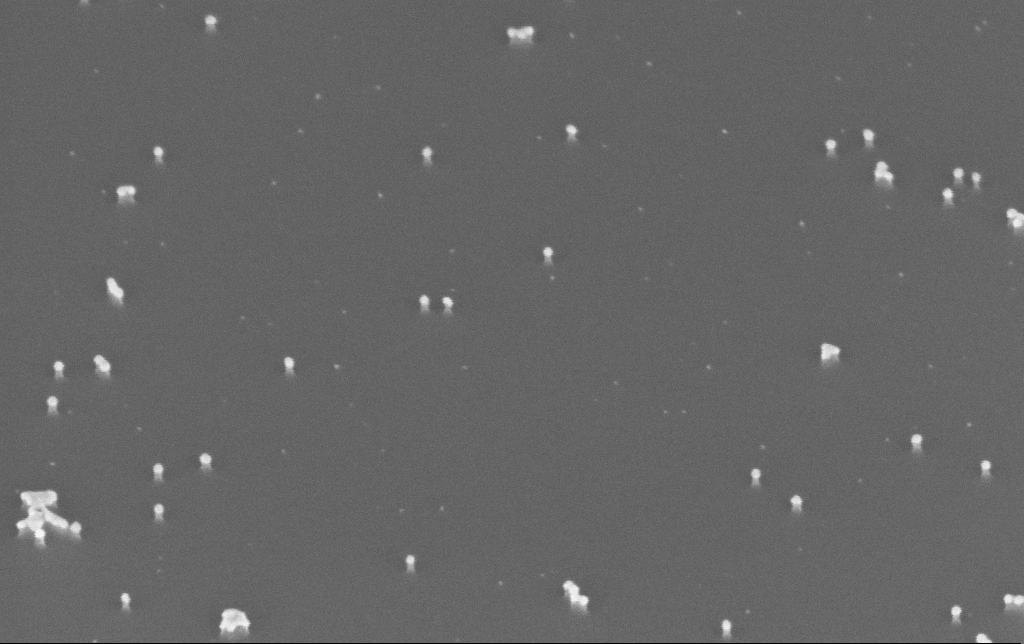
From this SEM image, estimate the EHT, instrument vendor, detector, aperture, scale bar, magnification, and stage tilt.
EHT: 10 kV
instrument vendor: Zeiss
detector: InLens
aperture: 30 µm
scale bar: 100 nm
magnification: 200 K X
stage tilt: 45°